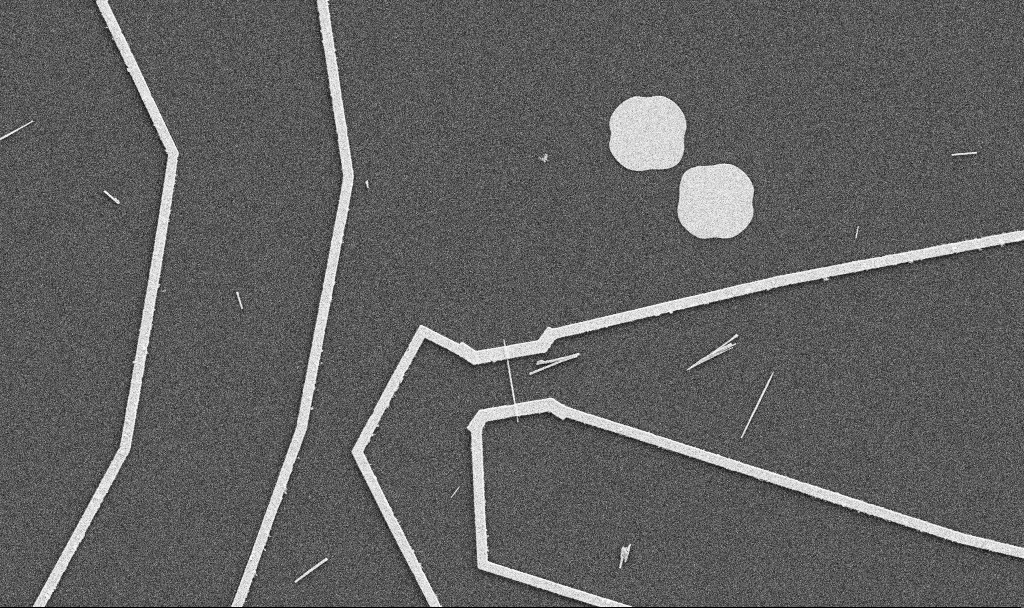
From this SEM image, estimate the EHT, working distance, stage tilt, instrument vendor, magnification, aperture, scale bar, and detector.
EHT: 5 kV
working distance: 10.7 mm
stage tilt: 0°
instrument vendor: Zeiss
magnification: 5 K X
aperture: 30 µm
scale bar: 10000 nm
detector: SE2